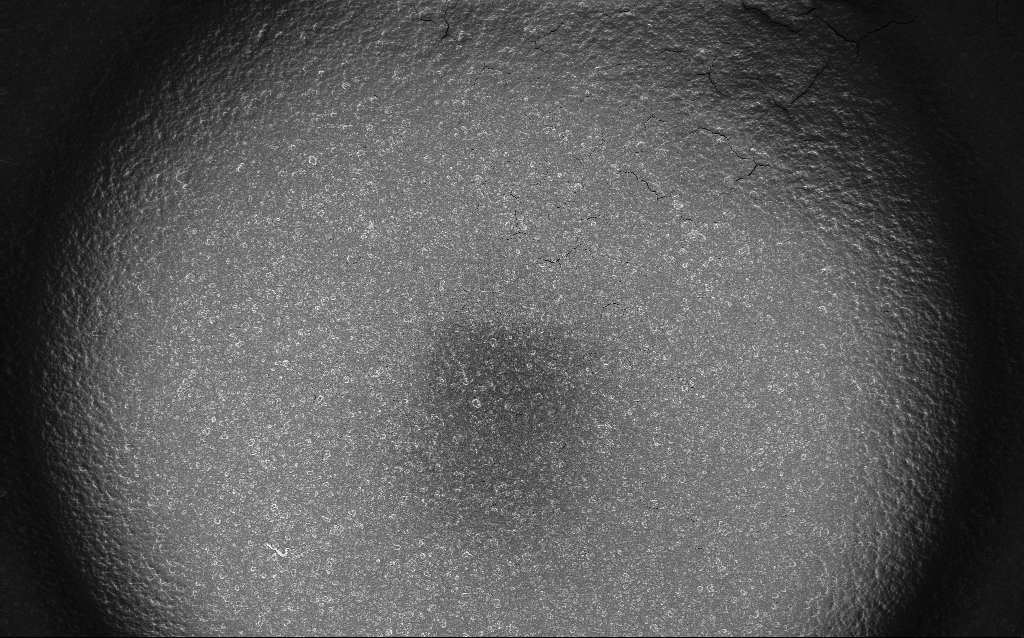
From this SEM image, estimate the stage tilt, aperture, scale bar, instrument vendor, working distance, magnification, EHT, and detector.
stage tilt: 0°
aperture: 30 µm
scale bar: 100000 nm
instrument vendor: Zeiss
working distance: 2.7 mm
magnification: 0.122 K X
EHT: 10 kV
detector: InLens